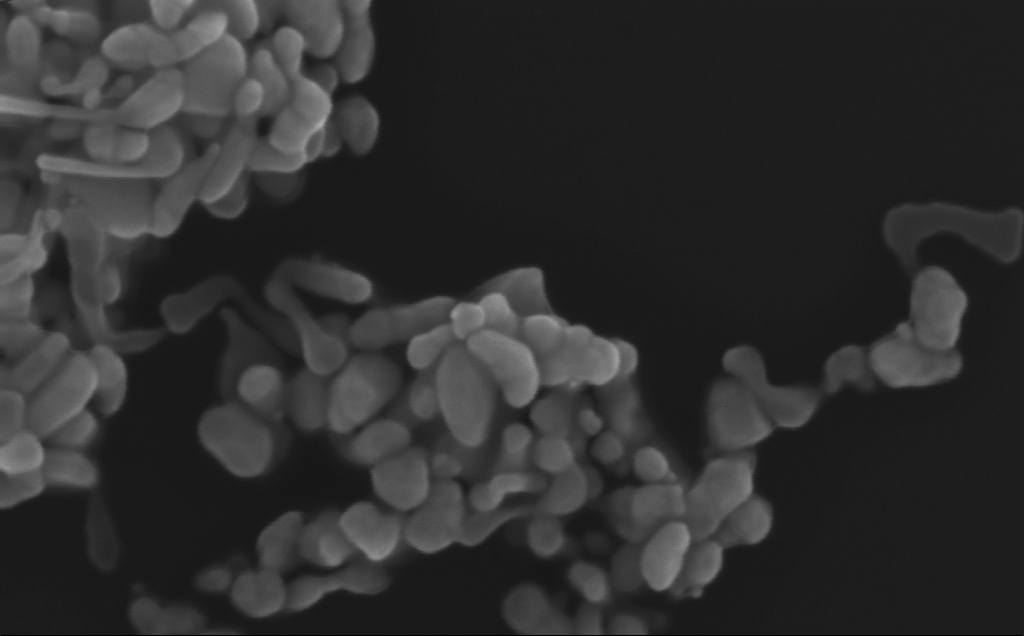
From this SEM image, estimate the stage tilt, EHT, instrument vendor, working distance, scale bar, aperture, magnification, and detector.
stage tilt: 0°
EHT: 10 kV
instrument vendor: Zeiss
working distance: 3 mm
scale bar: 200 nm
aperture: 30 µm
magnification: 331.92 K X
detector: InLens